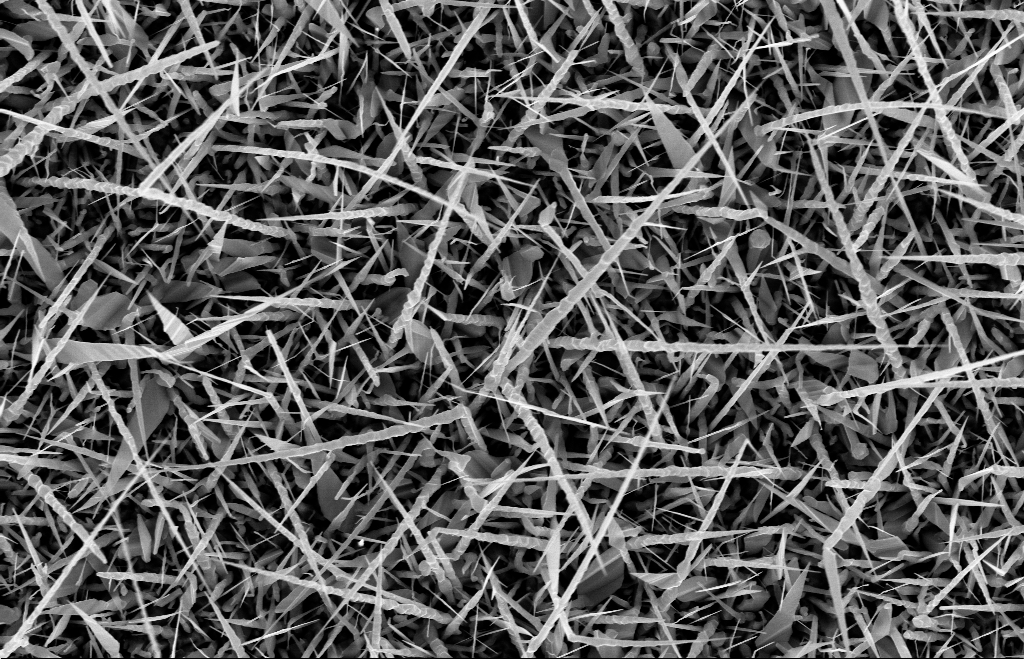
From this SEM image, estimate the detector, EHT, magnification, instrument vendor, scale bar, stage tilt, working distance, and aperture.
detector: InLens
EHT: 10 kV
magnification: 20 K X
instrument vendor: Zeiss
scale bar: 1000 nm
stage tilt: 0°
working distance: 9 mm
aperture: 30 µm